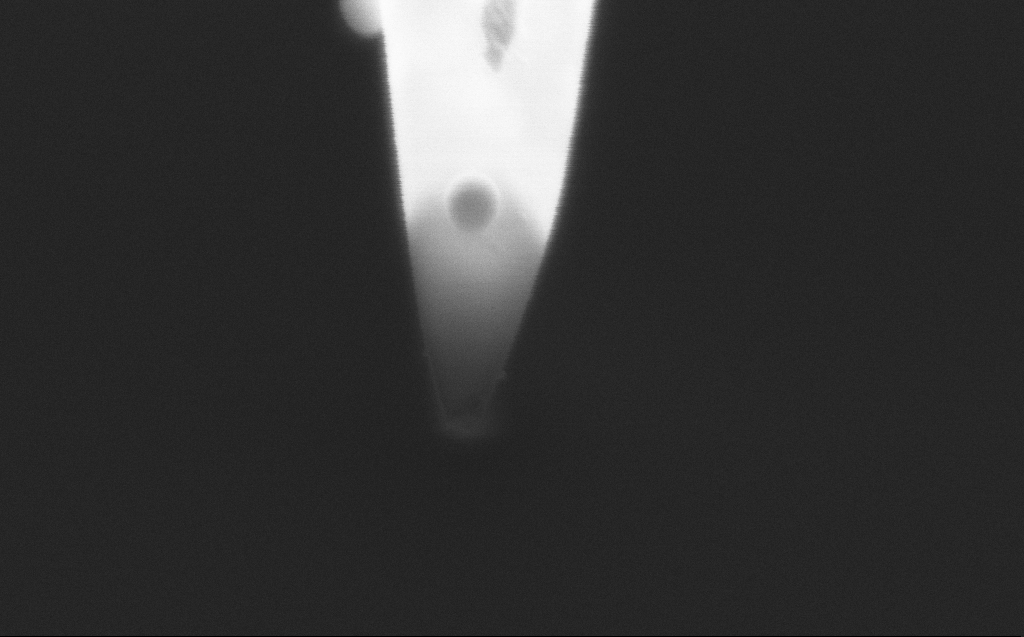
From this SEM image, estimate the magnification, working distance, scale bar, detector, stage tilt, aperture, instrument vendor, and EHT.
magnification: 196.18 K X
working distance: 4 mm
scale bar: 100 nm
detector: InLens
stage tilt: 45.1°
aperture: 20 µm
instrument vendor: Zeiss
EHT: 2 kV